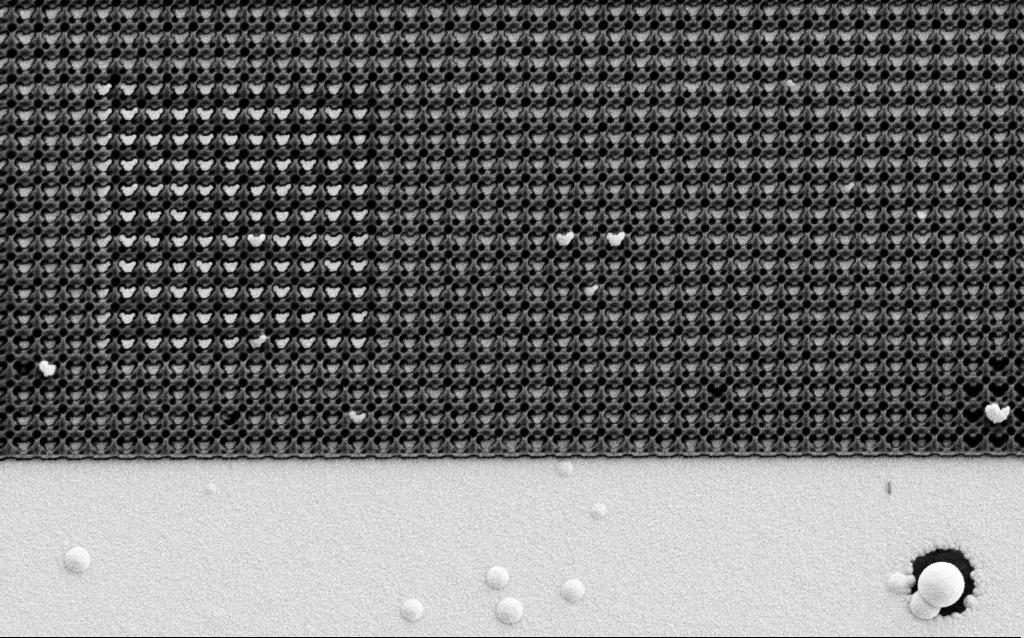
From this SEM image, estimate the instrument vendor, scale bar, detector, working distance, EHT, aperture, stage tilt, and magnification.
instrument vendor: Zeiss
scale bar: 2000 nm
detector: SE2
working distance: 8 mm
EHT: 1.5 kV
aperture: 30 µm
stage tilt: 0°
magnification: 20.17 K X